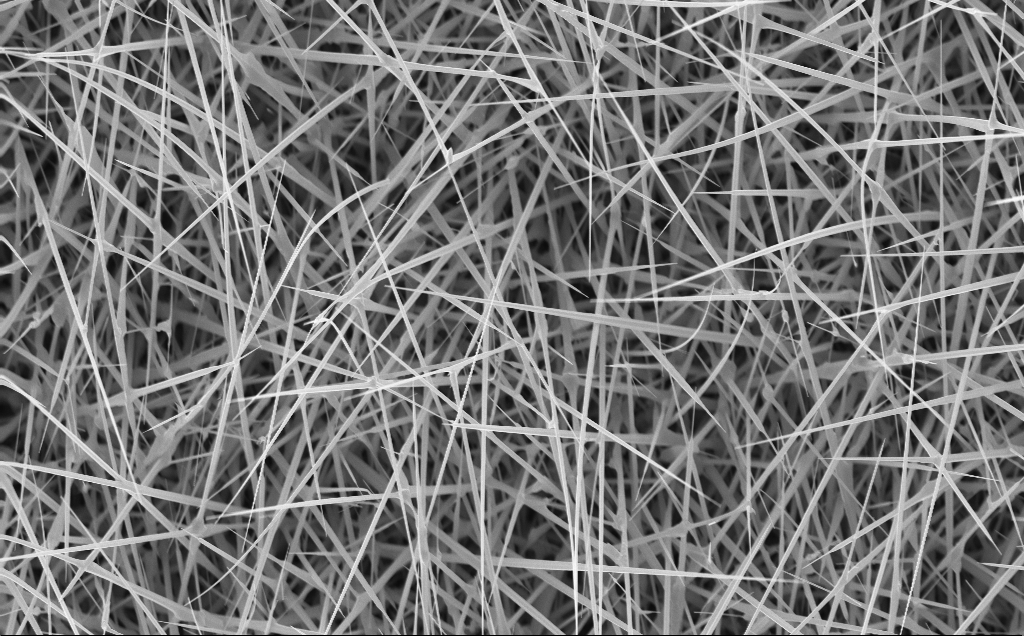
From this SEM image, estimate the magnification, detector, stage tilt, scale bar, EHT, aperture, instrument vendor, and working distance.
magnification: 10 K X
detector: InLens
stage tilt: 0°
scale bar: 2000 nm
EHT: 10 kV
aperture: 30 µm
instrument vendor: Zeiss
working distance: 4 mm